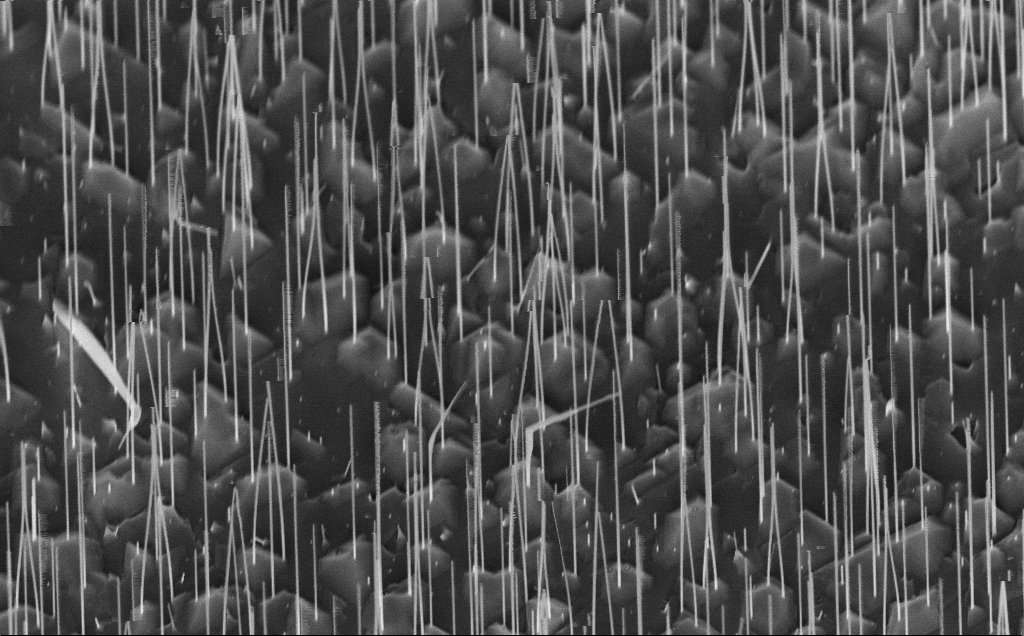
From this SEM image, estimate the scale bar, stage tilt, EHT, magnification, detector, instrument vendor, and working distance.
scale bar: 2000 nm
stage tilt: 45°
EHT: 10 kV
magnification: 27.97 K X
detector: InLens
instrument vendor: Zeiss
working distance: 7 mm